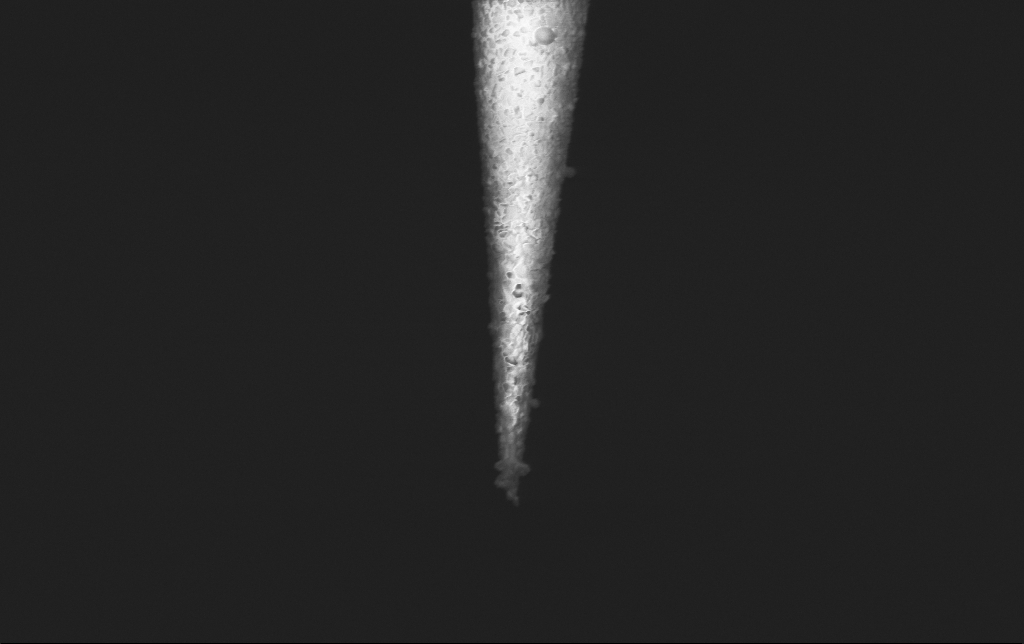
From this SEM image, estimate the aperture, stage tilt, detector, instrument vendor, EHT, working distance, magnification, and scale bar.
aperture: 30 µm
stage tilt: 0°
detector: InLens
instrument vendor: Zeiss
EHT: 2 kV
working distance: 6 mm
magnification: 50 K X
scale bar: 1000 nm